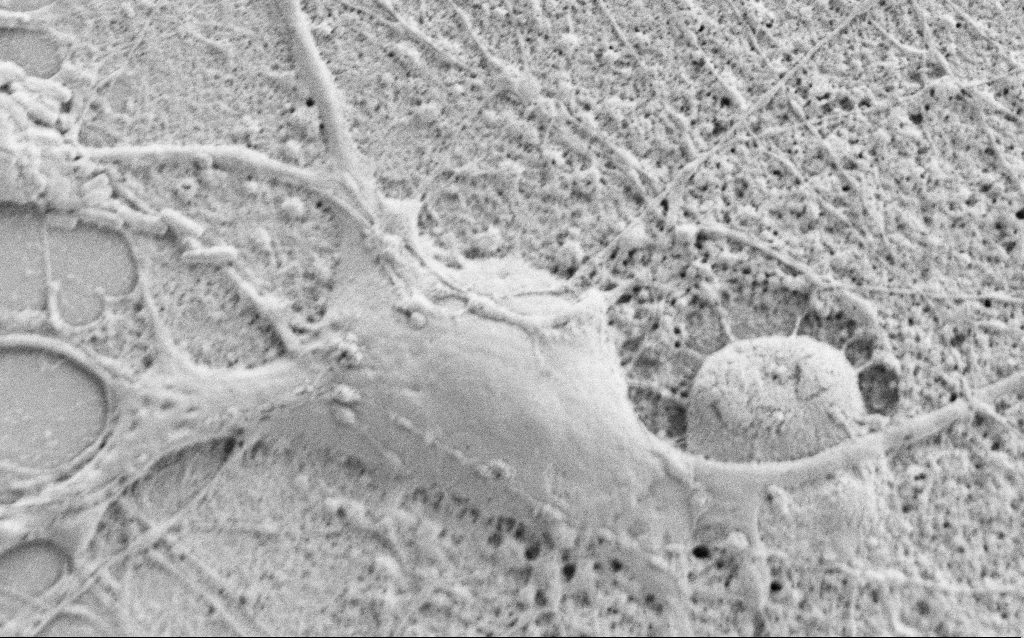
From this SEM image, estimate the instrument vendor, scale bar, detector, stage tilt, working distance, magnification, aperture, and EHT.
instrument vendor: Zeiss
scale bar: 2000 nm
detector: SE2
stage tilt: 0°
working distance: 5.7 mm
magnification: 7.5 K X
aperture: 30 µm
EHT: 0.8 kV